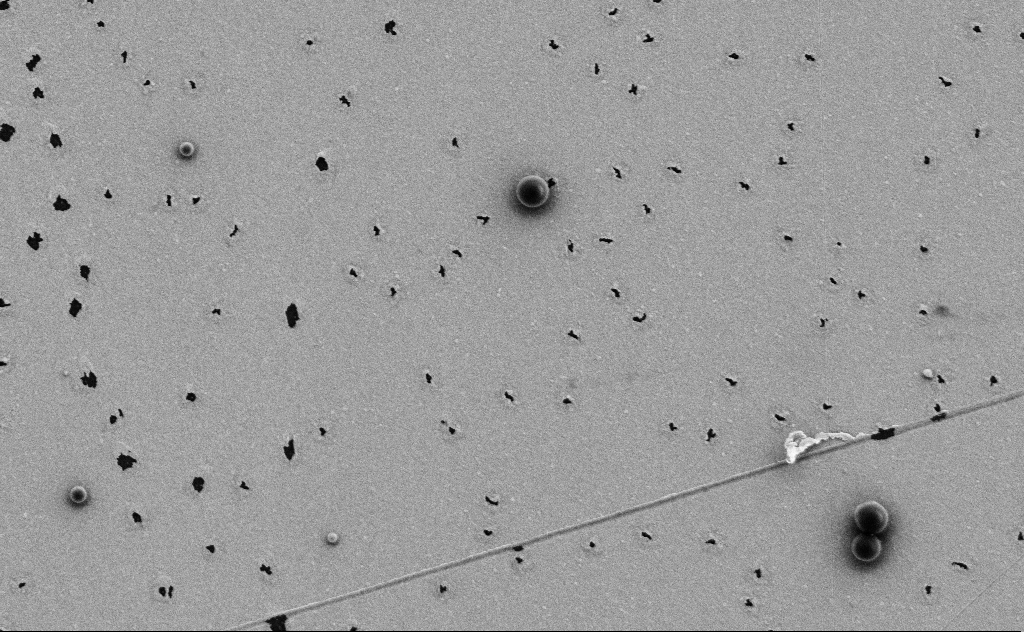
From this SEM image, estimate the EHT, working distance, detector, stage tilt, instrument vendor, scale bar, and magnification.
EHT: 3 kV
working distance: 10 mm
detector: SE2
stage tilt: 0°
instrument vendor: Zeiss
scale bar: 2000 nm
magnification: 13.65 K X